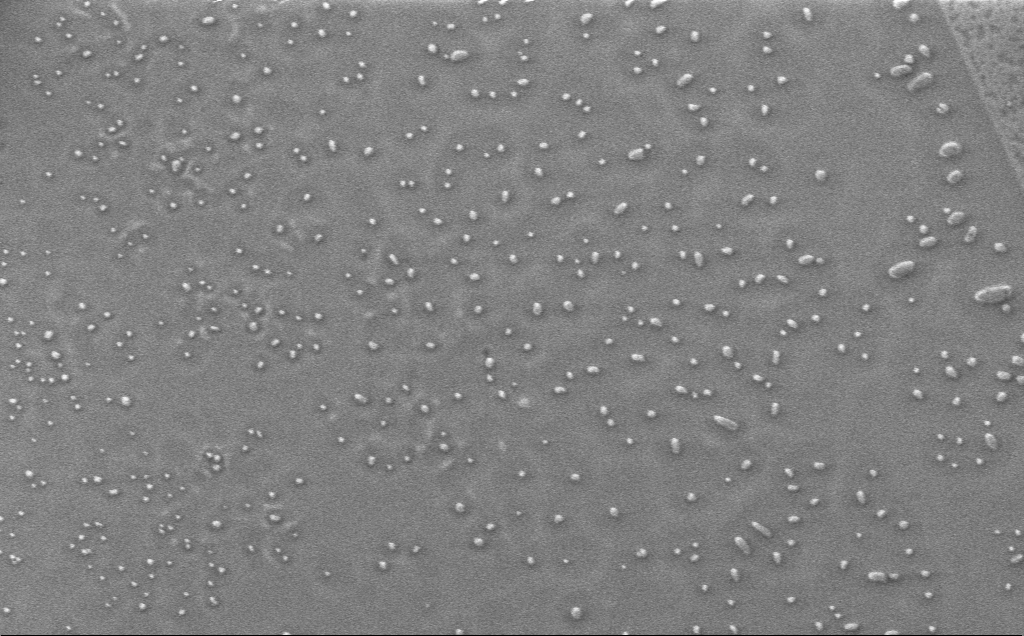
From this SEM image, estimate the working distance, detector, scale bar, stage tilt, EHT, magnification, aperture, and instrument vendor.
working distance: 3 mm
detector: InLens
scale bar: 10000 nm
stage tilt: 0°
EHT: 1 kV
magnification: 4.86 K X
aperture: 30 µm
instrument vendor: Zeiss